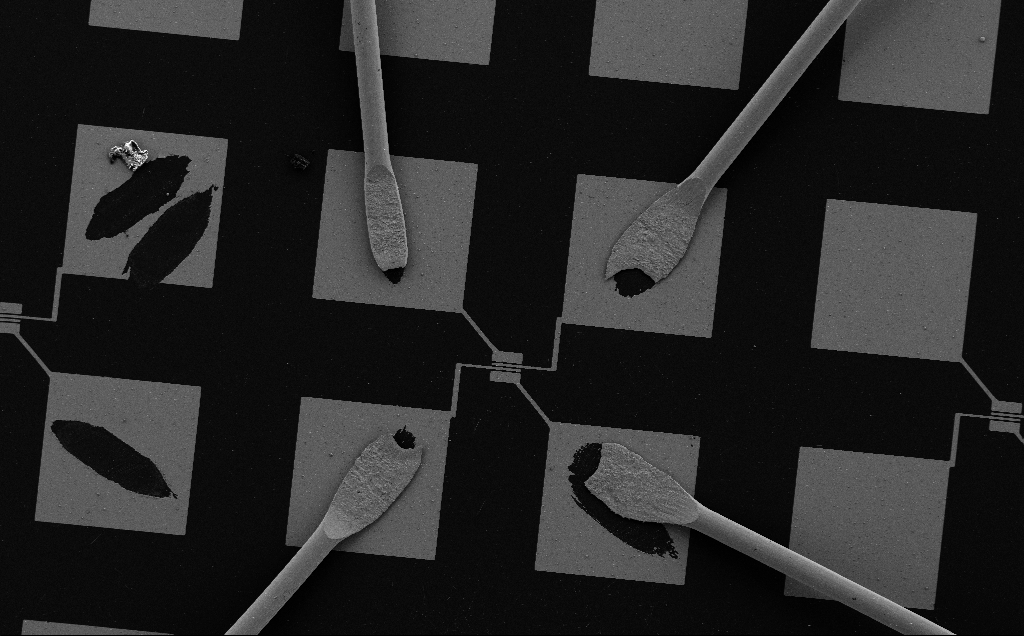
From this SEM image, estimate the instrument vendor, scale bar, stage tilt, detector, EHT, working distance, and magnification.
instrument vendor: Zeiss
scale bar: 100000 nm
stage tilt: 0°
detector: SE2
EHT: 5 kV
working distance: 8 mm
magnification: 0.371 K X